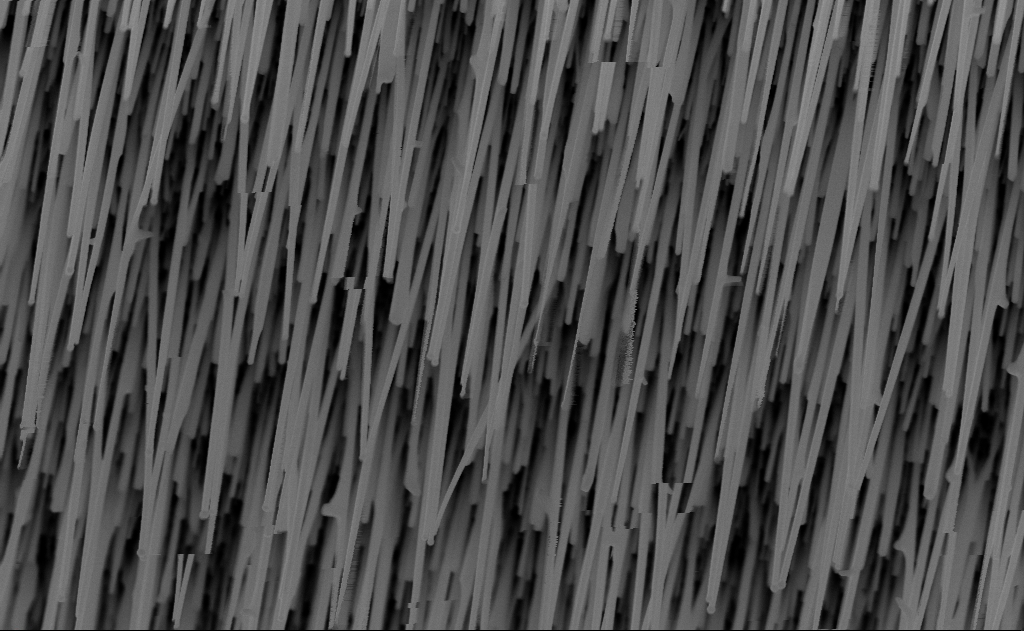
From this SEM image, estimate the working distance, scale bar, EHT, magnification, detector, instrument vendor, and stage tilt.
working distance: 9 mm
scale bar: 2000 nm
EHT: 10 kV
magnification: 30 K X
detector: InLens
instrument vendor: Zeiss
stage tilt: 0°